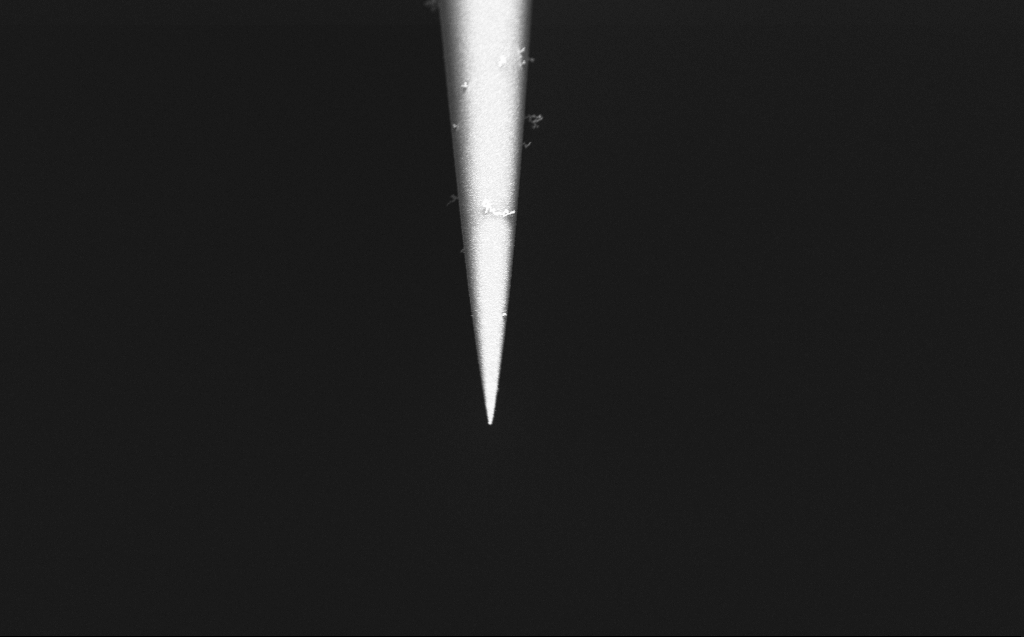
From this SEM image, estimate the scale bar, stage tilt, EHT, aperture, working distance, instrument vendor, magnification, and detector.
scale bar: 2000 nm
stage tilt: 45°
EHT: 2 kV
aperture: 30 µm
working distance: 4 mm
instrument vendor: Zeiss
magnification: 10 K X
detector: InLens